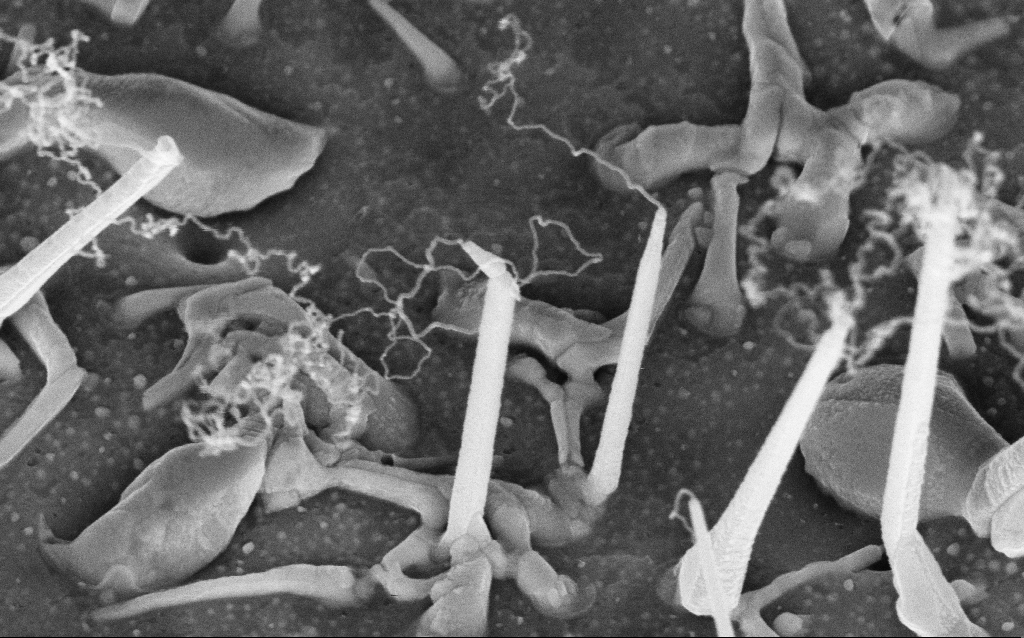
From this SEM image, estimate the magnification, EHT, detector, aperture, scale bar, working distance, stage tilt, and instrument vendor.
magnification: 106.66 K X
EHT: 5 kV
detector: InLens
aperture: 30 µm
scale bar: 200 nm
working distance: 8 mm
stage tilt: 42°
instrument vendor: Zeiss